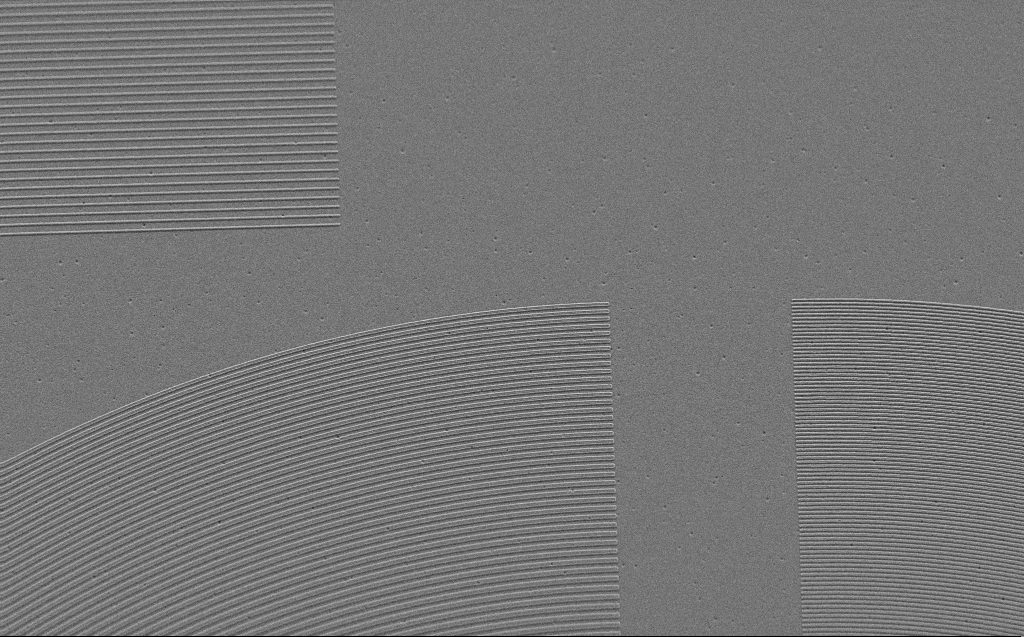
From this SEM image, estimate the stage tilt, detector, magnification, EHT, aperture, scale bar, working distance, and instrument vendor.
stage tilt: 29.8°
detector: SE2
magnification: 6.8 K X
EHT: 2 kV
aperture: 30 µm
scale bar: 10000 nm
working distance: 4 mm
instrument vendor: Zeiss